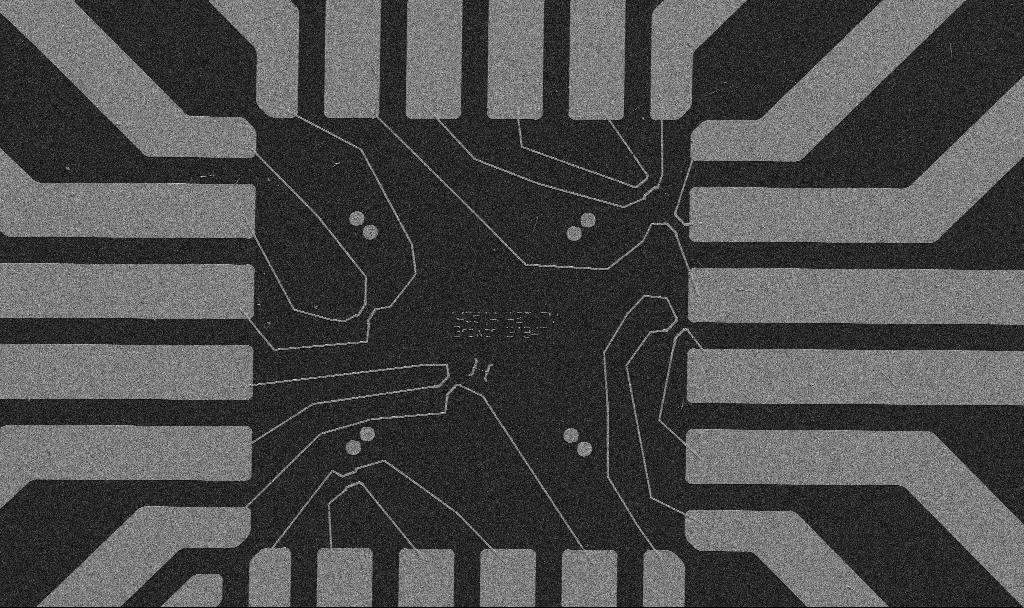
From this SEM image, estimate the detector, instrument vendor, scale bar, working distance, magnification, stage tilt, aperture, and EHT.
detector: SE2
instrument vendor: Zeiss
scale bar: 20000 nm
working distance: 10.7 mm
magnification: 1 K X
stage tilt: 0°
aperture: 30 µm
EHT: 5 kV